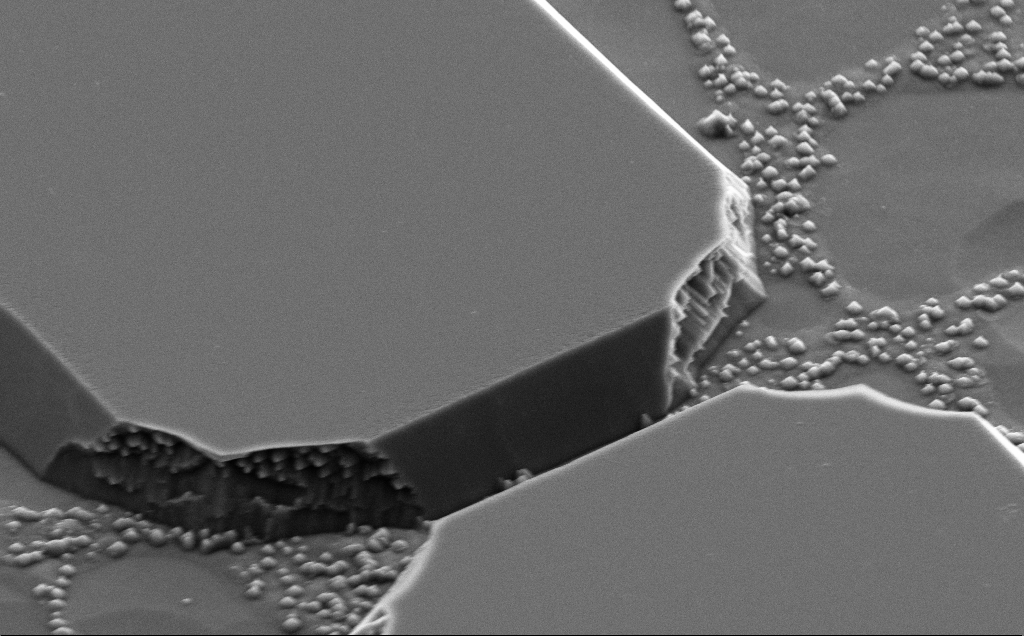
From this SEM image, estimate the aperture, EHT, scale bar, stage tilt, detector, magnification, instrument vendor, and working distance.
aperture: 30 µm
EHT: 5 kV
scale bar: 1000 nm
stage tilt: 50°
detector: SE2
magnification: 14.46 K X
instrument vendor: Zeiss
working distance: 10 mm